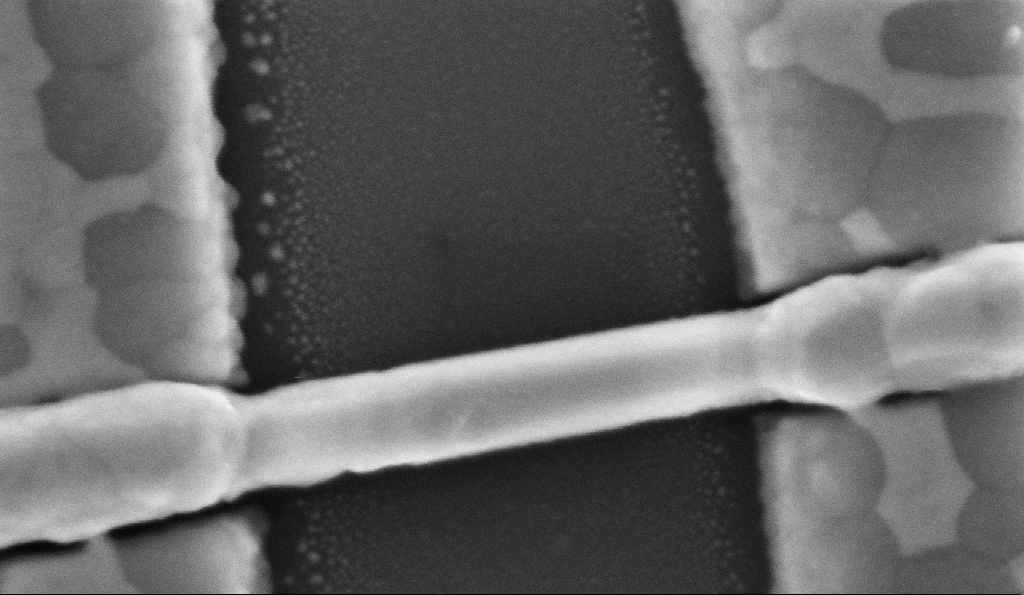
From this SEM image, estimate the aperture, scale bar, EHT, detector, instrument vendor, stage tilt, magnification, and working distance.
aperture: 30 µm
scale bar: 200 nm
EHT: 5 kV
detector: InLens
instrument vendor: Zeiss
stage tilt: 0°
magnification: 316.02 K X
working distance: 8.6 mm